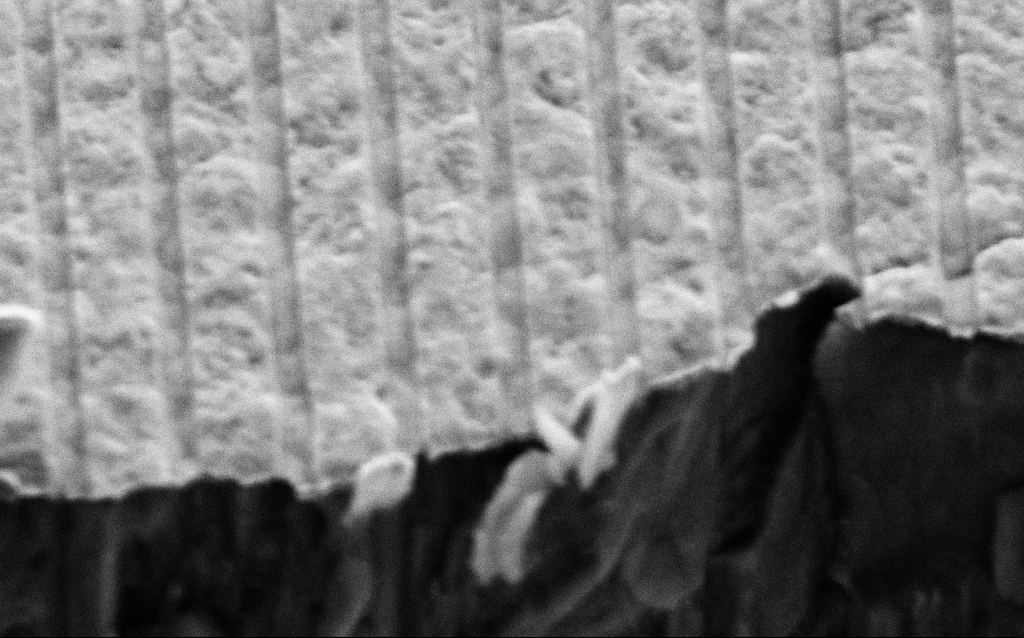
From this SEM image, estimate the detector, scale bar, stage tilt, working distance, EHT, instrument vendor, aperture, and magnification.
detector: SE2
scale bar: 200 nm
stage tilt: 45°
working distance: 6 mm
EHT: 3 kV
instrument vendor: Zeiss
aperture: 30 µm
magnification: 83.2 K X